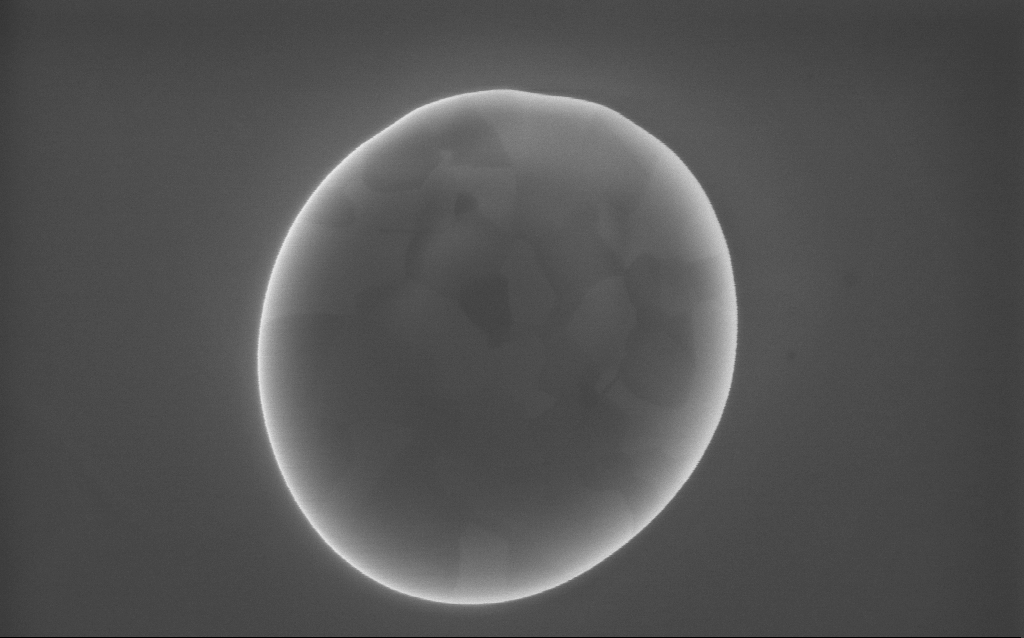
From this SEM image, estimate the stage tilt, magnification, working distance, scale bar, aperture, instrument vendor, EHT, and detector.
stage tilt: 0°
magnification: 97 K X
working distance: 2 mm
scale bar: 200 nm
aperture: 30 µm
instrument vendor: Zeiss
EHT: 10 kV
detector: InLens